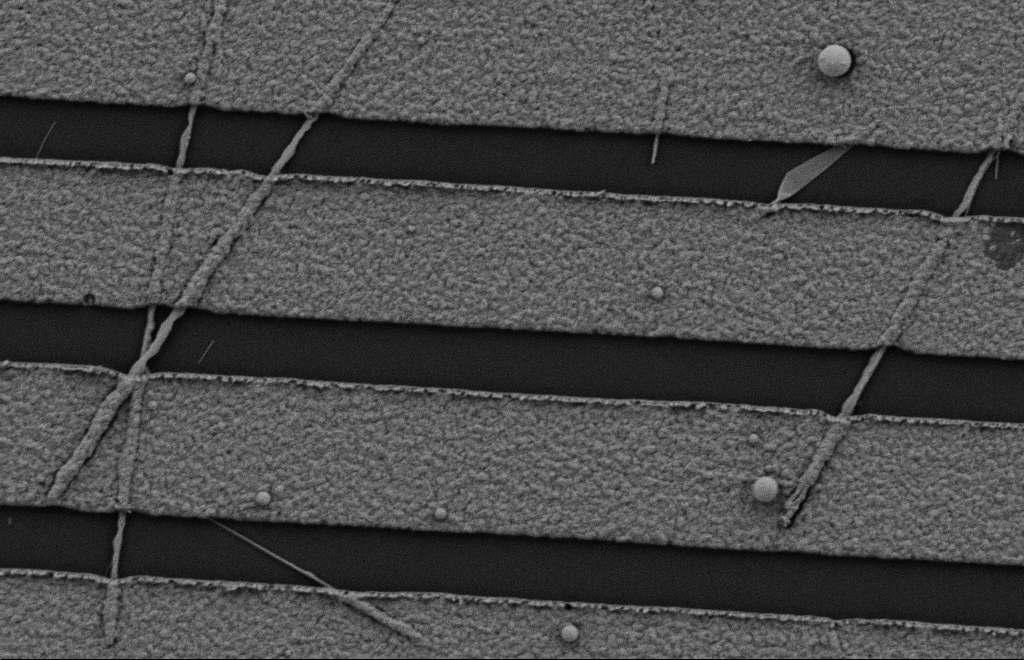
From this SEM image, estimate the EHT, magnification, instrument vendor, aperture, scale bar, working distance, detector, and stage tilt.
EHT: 2 kV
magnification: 18.73 K X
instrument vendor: Zeiss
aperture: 20 µm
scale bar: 2000 nm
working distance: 10 mm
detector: SE2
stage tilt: -0.3°